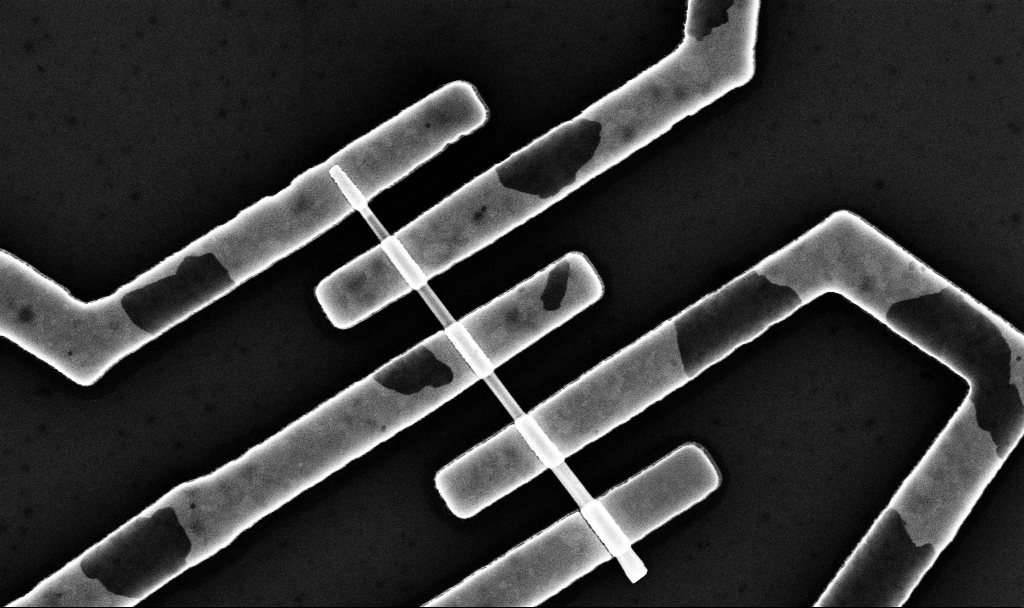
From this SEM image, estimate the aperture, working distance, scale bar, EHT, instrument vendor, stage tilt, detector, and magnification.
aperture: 30 µm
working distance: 6.7 mm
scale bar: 1000 nm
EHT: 10 kV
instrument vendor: Zeiss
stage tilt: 0°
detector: InLens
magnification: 35.23 K X